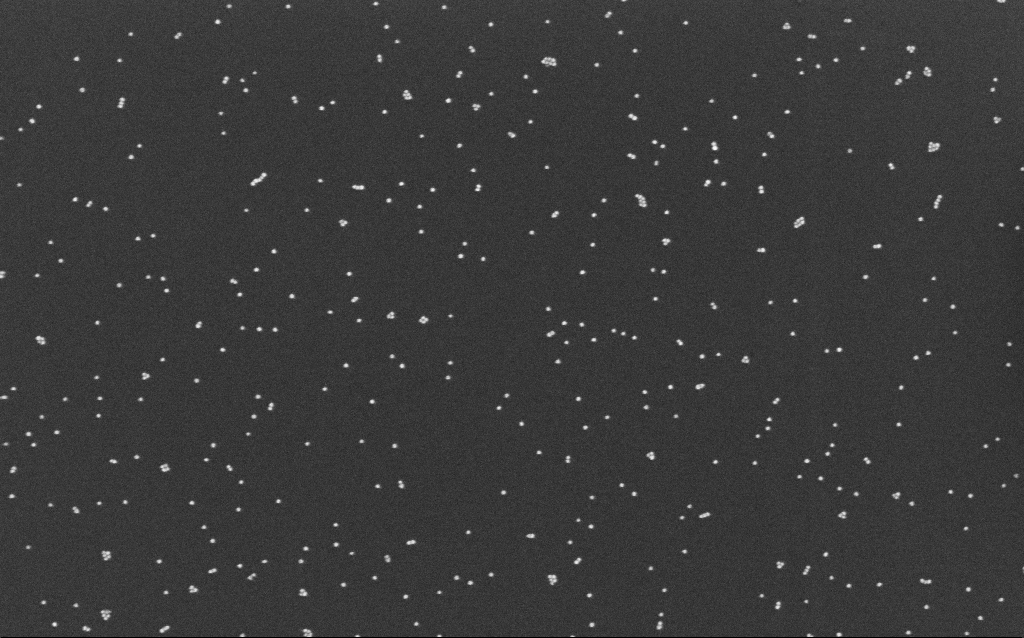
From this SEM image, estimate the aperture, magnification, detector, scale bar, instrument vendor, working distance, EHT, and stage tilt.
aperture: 30 µm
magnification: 100 K X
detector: InLens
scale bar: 200 nm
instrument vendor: Zeiss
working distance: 6.5 mm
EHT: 10 kV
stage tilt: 0°